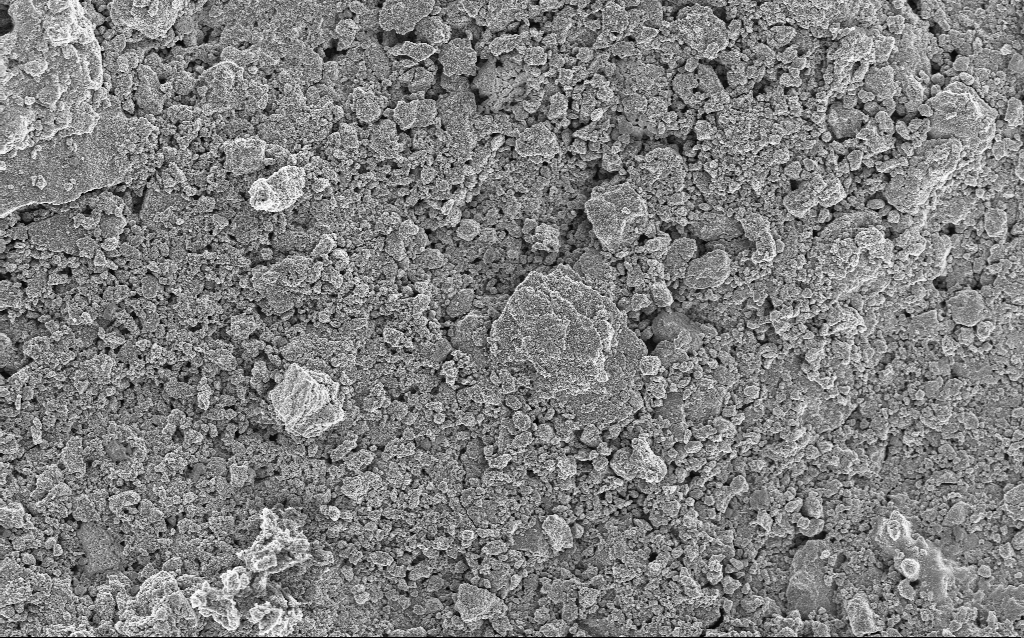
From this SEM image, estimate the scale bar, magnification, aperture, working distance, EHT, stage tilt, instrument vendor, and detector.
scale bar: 10000 nm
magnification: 1.23 K X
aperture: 30 µm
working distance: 4.5 mm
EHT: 5 kV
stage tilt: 0°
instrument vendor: Zeiss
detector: InLens